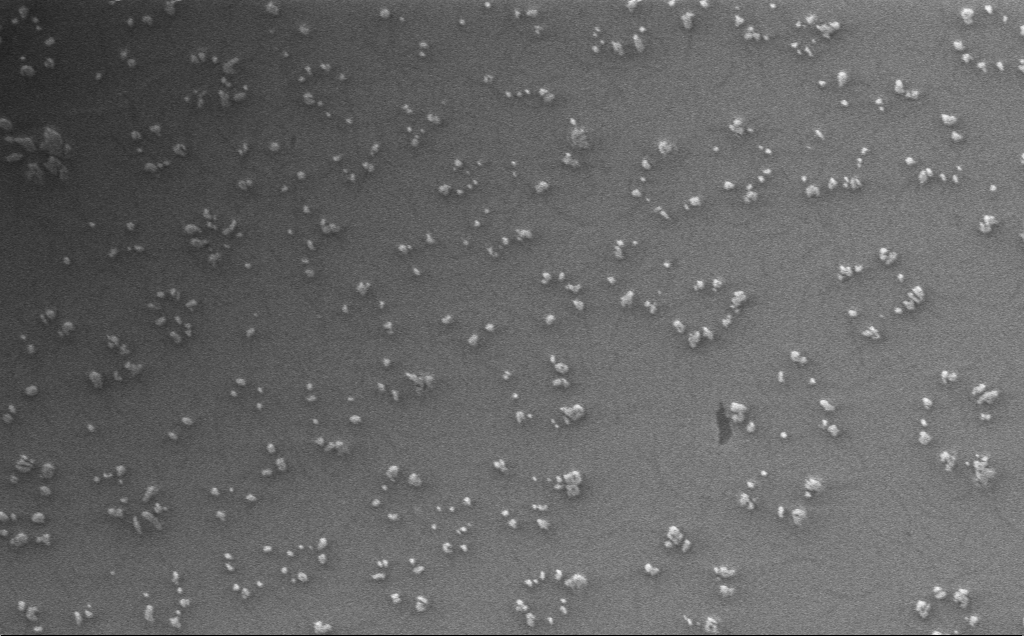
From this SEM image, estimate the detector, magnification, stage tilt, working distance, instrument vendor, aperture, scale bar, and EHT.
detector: InLens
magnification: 3.37 K X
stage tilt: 0°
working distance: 3 mm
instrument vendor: Zeiss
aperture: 30 µm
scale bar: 20000 nm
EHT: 1 kV